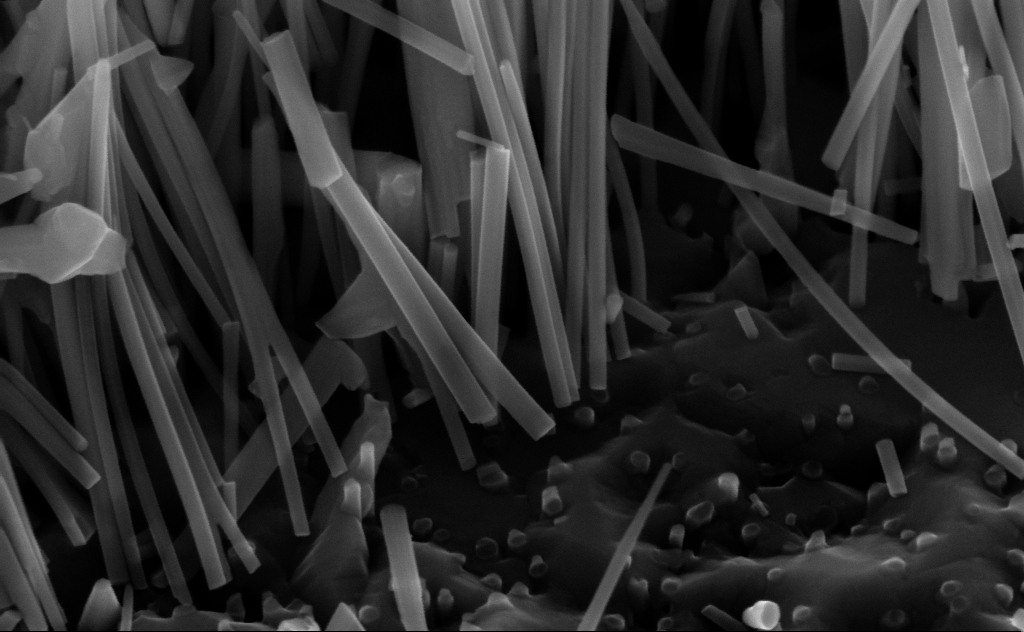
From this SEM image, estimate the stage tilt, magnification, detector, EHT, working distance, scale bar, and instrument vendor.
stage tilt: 45°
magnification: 159.6 K X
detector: InLens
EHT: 10 kV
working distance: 6 mm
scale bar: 100 nm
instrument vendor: Zeiss